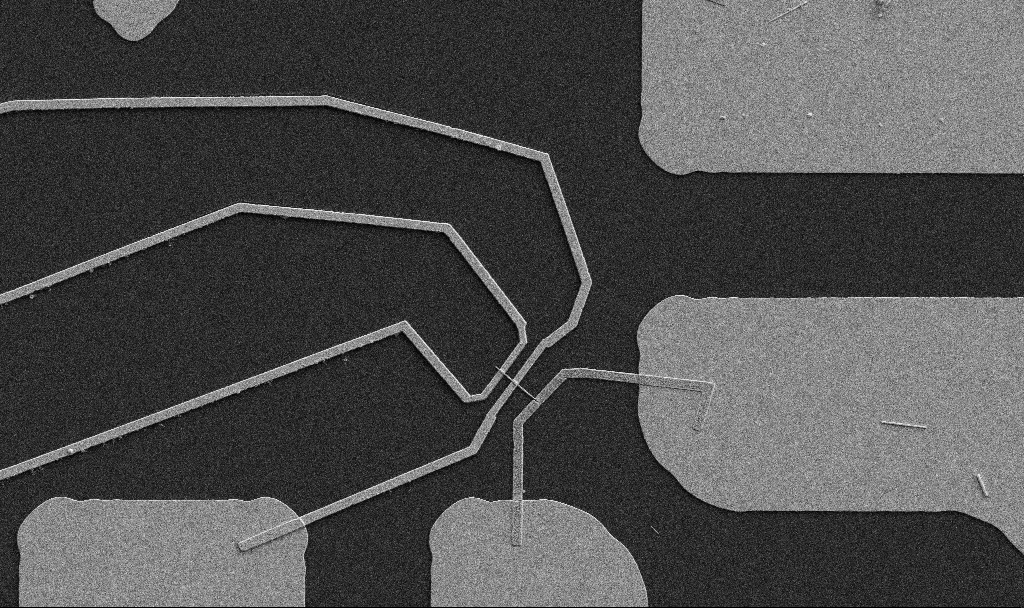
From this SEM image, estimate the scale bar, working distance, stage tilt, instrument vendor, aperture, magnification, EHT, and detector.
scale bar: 10000 nm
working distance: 10.7 mm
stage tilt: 0°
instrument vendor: Zeiss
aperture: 30 µm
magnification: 5 K X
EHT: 5 kV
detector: SE2